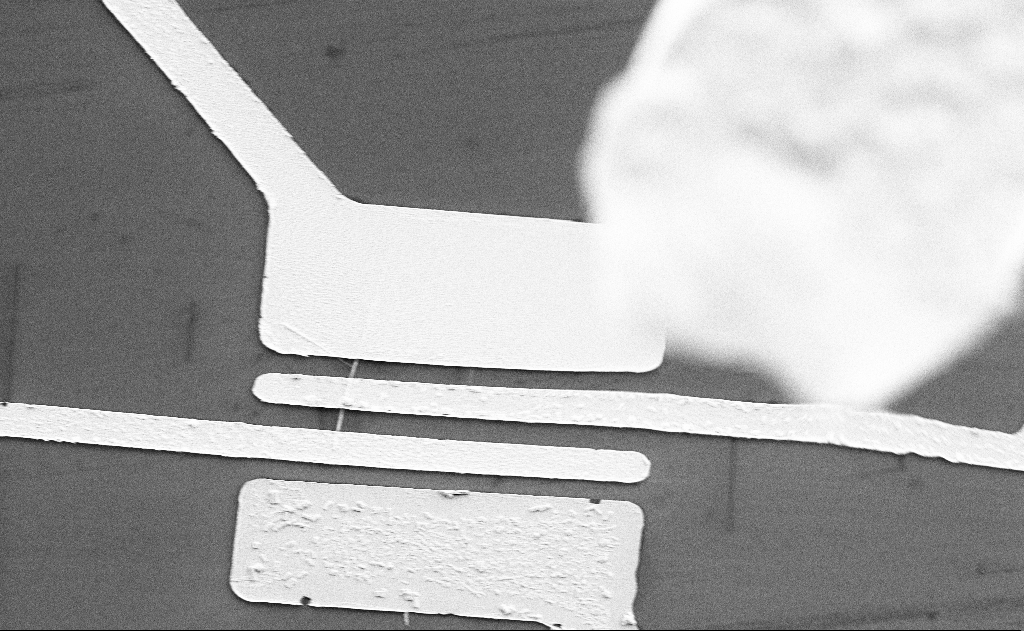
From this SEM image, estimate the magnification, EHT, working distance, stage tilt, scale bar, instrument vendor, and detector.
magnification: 5 K X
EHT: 5 kV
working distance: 15 mm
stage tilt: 0°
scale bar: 10000 nm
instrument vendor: Zeiss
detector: SE2